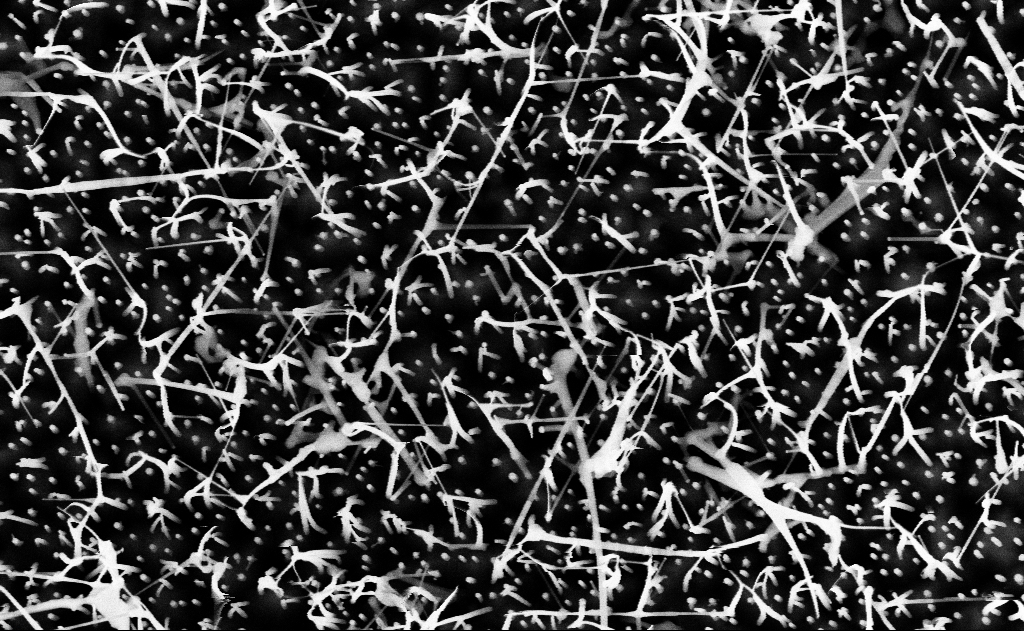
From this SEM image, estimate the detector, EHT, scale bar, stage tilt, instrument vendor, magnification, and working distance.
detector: InLens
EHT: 10 kV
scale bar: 1000 nm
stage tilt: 0°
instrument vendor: Zeiss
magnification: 40 K X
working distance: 16 mm